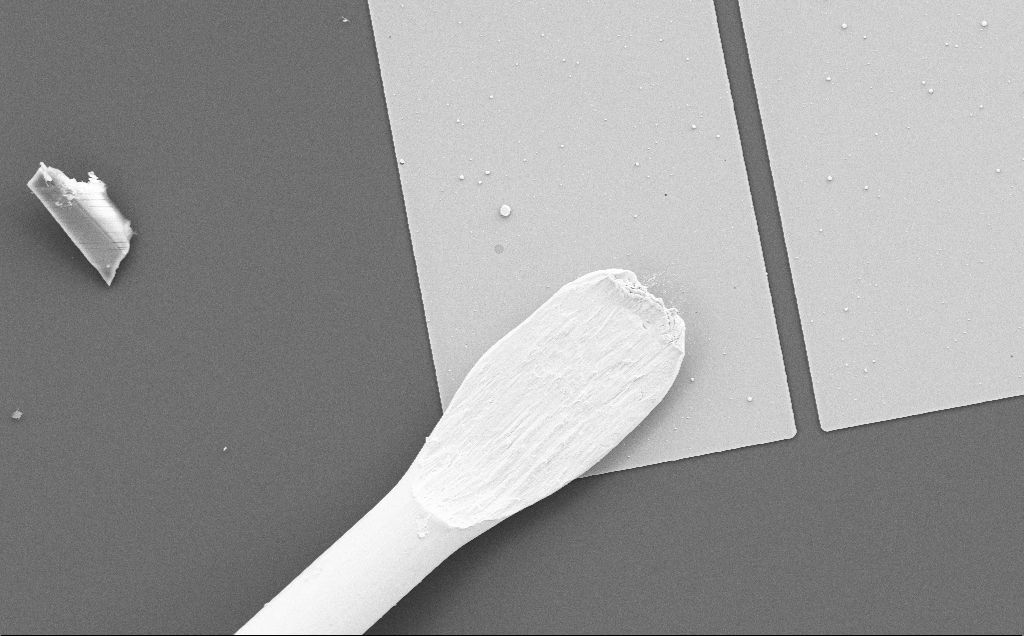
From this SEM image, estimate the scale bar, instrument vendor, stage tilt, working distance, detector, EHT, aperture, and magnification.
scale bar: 20000 nm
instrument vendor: Zeiss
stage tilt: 0°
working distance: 11 mm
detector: SE2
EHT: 15 kV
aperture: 30 µm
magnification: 1.3 K X